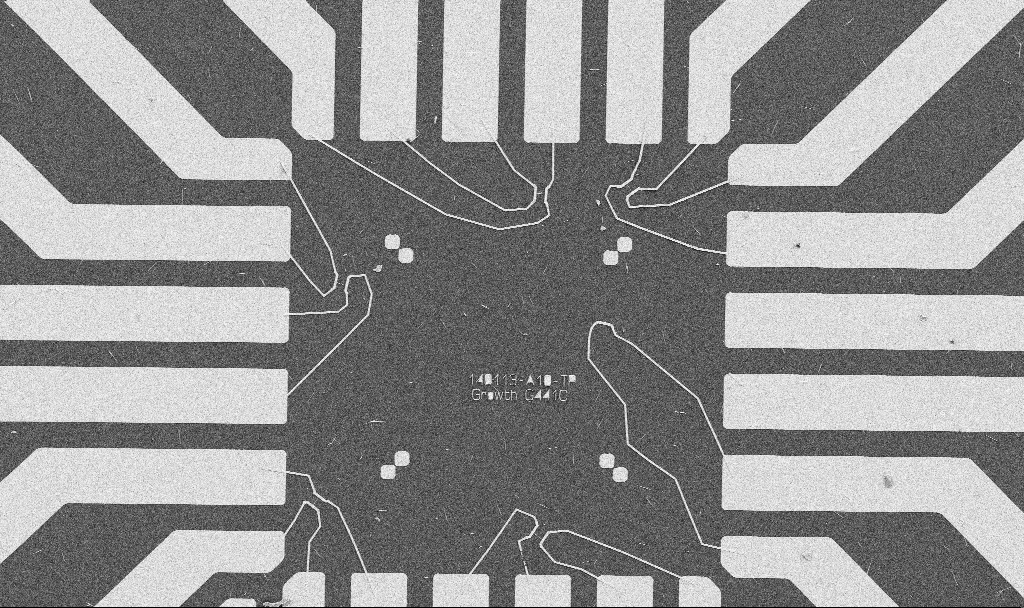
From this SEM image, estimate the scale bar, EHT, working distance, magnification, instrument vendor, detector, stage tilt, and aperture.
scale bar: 20000 nm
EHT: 5 kV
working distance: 10.7 mm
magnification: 1 K X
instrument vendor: Zeiss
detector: SE2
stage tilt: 0°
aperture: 30 µm